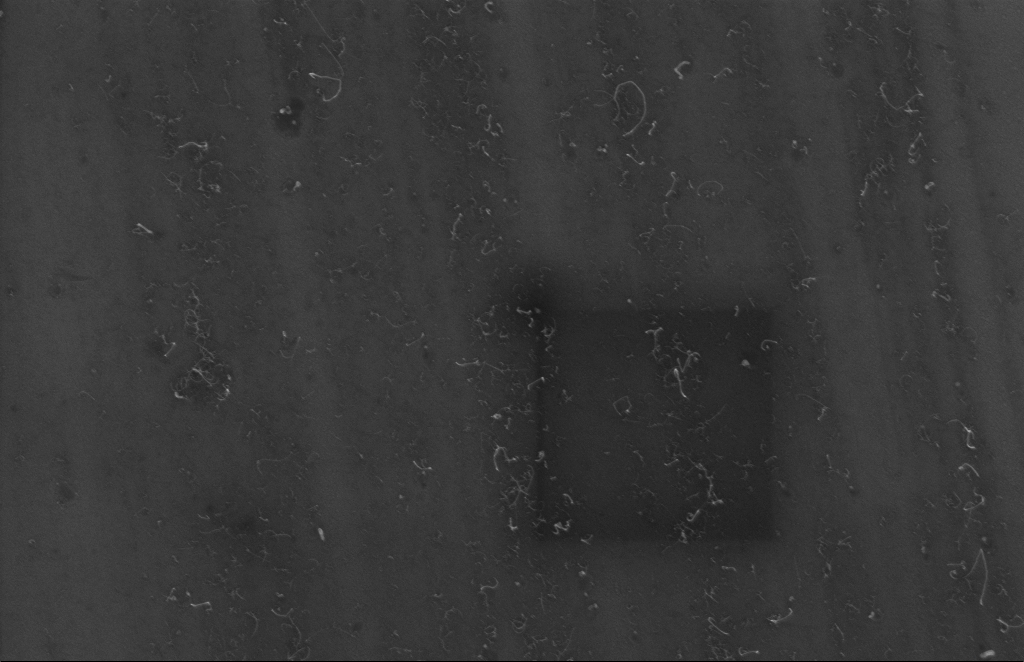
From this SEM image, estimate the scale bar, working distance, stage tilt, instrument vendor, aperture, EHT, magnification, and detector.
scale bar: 200 nm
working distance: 5 mm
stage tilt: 0°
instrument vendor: Zeiss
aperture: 20 µm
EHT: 5 kV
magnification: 58.37 K X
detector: InLens